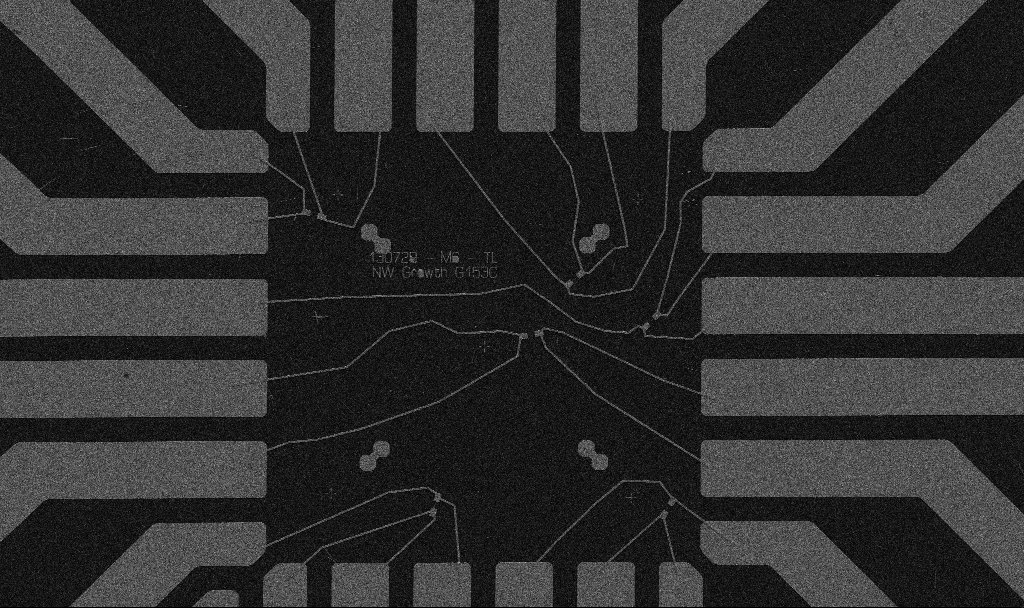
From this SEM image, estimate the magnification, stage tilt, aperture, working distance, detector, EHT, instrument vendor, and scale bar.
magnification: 1 K X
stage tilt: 0°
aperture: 30 µm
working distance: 10.7 mm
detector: SE2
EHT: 5 kV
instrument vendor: Zeiss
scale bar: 20000 nm